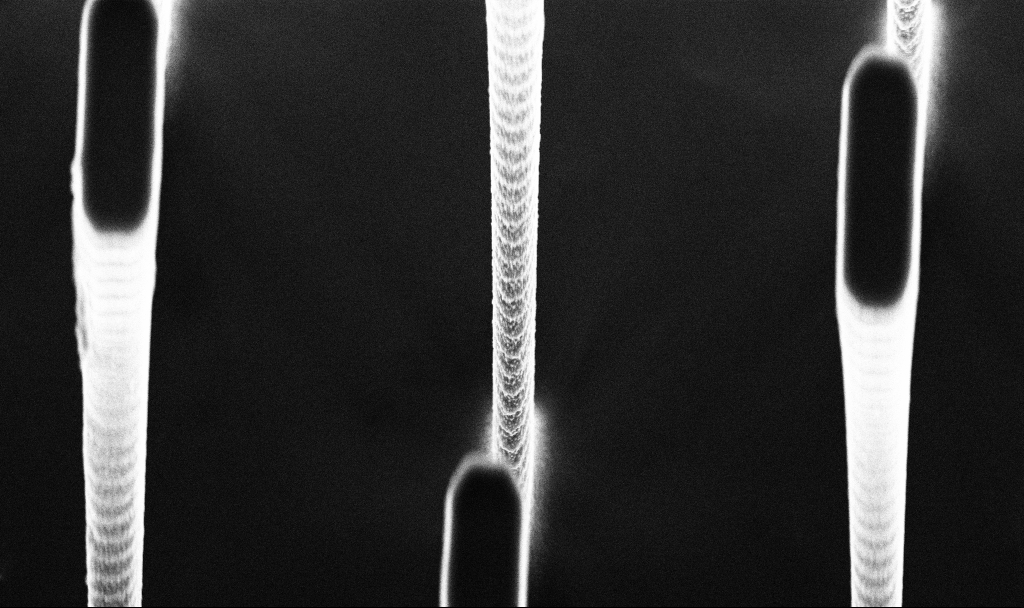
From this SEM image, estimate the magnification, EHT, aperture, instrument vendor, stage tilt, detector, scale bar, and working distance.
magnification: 15.6 K X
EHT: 5 kV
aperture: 30 µm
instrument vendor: Zeiss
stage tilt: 30°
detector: InLens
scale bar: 2000 nm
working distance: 6.6 mm